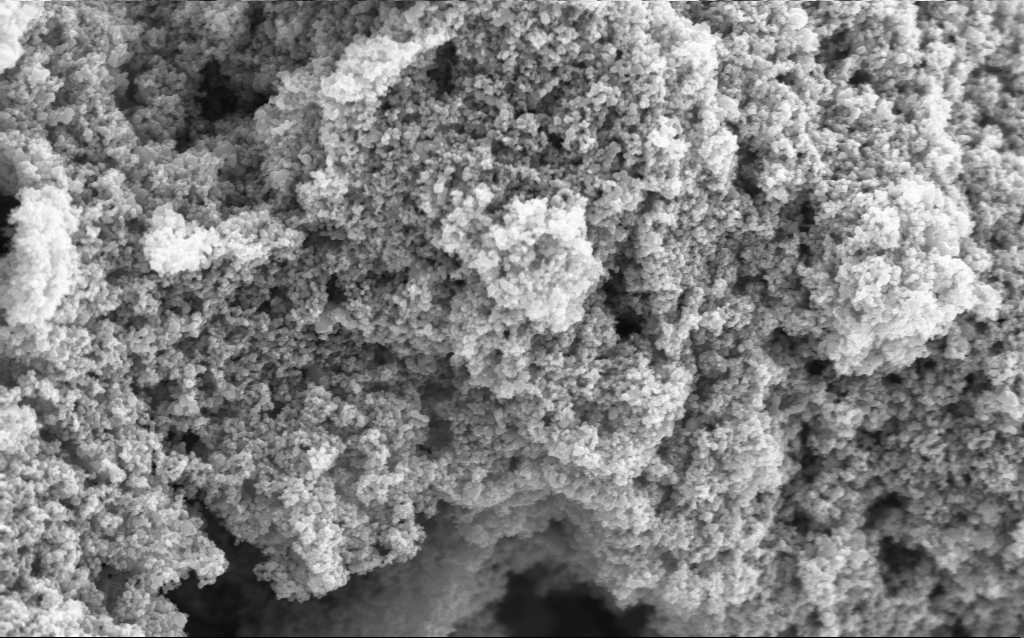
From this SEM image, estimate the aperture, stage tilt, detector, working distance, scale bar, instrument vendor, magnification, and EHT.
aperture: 30 µm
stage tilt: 0°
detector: InLens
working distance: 4.4 mm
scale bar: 1000 nm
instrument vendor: Zeiss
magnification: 68.66 K X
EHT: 5 kV